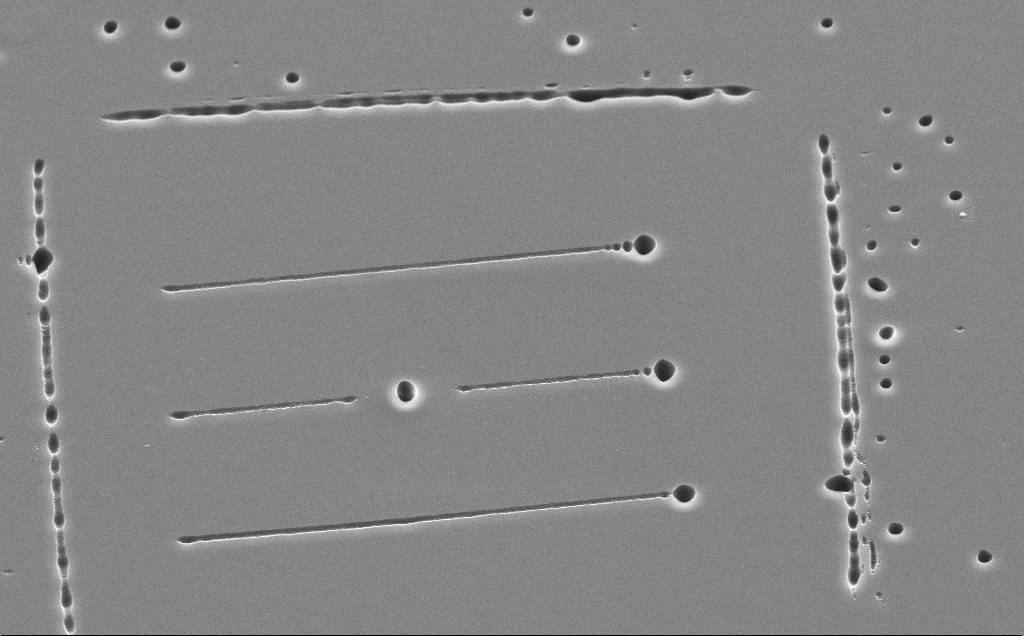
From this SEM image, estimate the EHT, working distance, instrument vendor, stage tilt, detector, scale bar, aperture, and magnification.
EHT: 10 kV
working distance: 12 mm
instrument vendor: Zeiss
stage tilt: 45°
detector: SE2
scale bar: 10000 nm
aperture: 30 µm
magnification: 3.23 K X